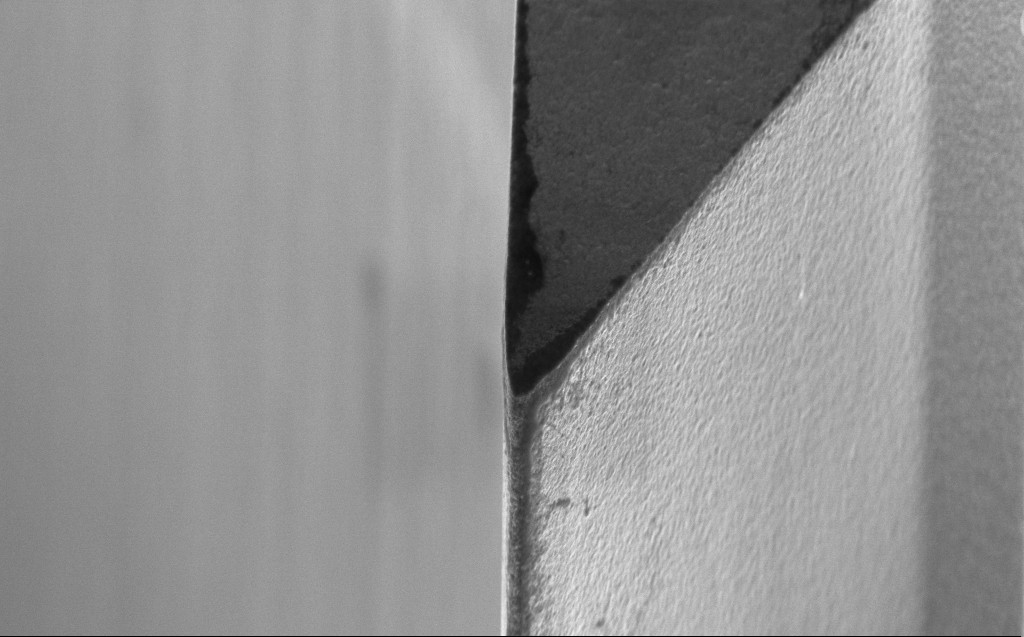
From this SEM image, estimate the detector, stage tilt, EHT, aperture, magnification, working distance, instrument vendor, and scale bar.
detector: InLens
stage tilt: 45°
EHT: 5 kV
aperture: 30 µm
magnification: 17.5 K X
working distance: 5 mm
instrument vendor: Zeiss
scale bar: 1000 nm